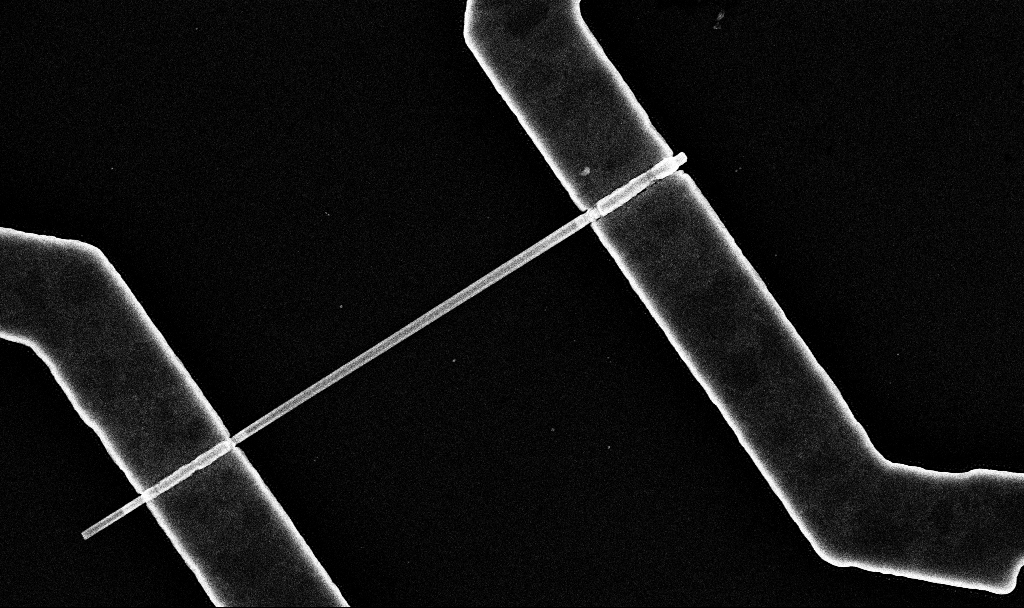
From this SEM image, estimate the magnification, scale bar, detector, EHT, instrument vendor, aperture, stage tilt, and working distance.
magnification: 44.02 K X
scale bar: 1000 nm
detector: InLens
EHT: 10 kV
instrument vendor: Zeiss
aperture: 30 µm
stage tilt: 0°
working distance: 6.7 mm